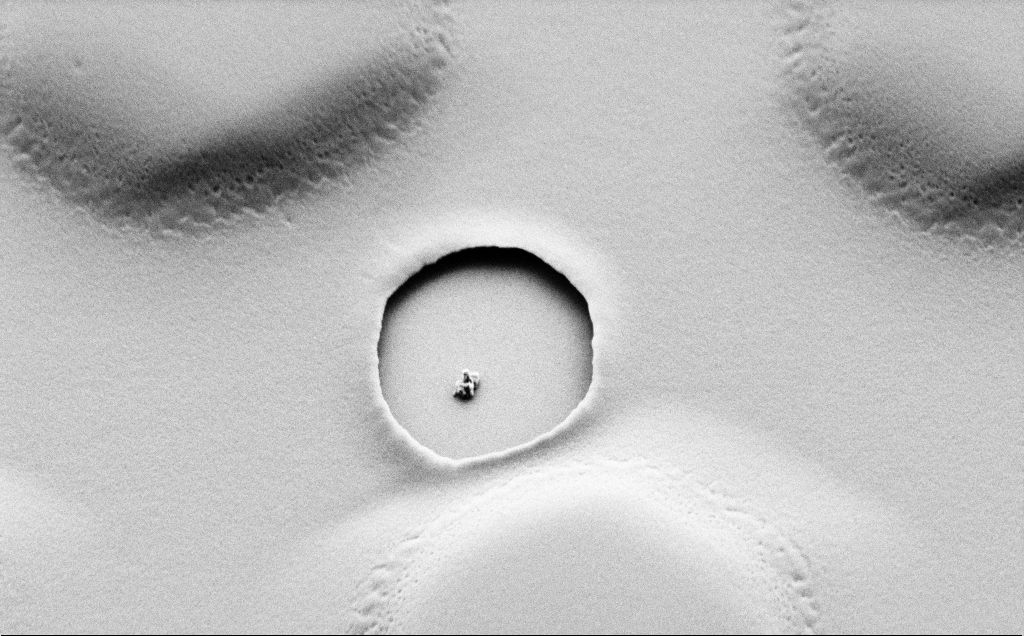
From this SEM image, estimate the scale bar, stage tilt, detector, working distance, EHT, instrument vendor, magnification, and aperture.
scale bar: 1000 nm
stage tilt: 45°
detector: SE2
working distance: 4 mm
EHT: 1.5 kV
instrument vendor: Zeiss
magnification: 15.36 K X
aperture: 30 µm